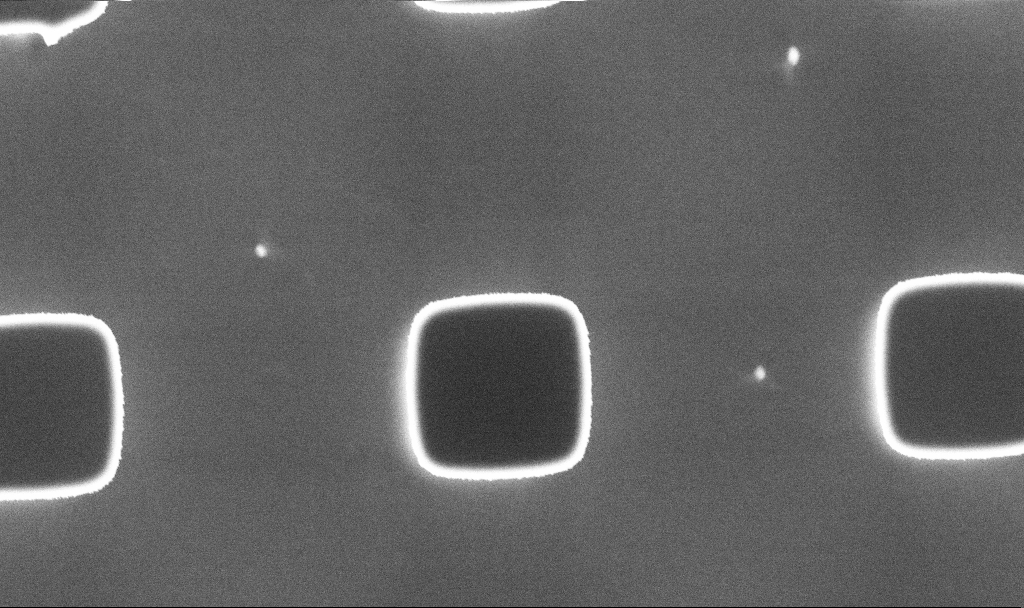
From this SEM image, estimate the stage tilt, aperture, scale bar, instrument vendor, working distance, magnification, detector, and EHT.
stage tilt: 0°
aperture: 30 µm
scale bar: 2000 nm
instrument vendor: Zeiss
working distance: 5.3 mm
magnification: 14.41 K X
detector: InLens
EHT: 5 kV